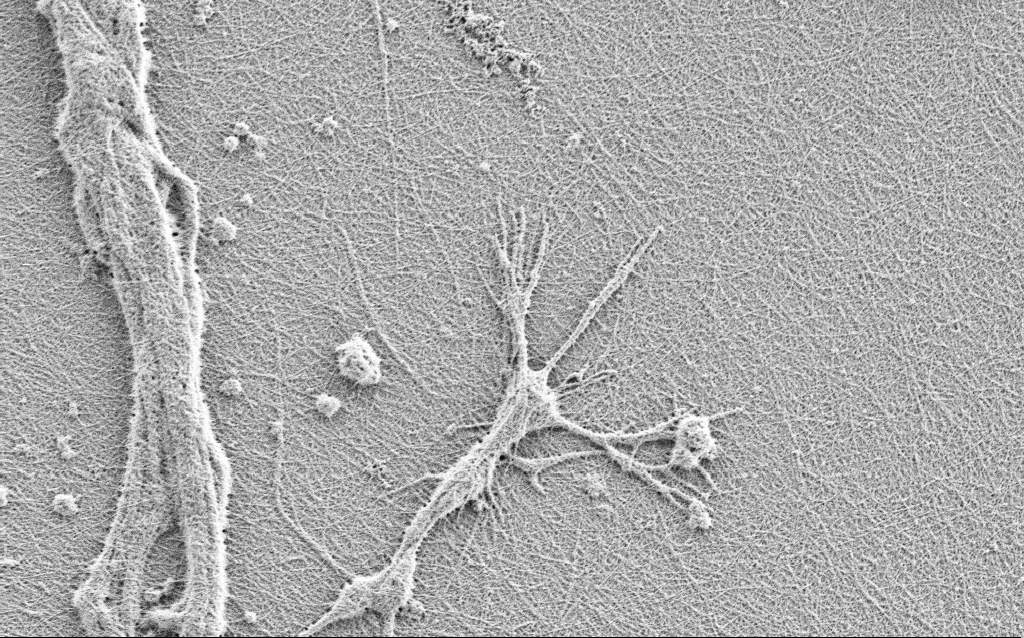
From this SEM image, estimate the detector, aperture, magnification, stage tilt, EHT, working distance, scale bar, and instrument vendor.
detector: SE2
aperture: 30 µm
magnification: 10 K X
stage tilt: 0°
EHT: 0.9 kV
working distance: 3 mm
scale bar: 2000 nm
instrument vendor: Zeiss